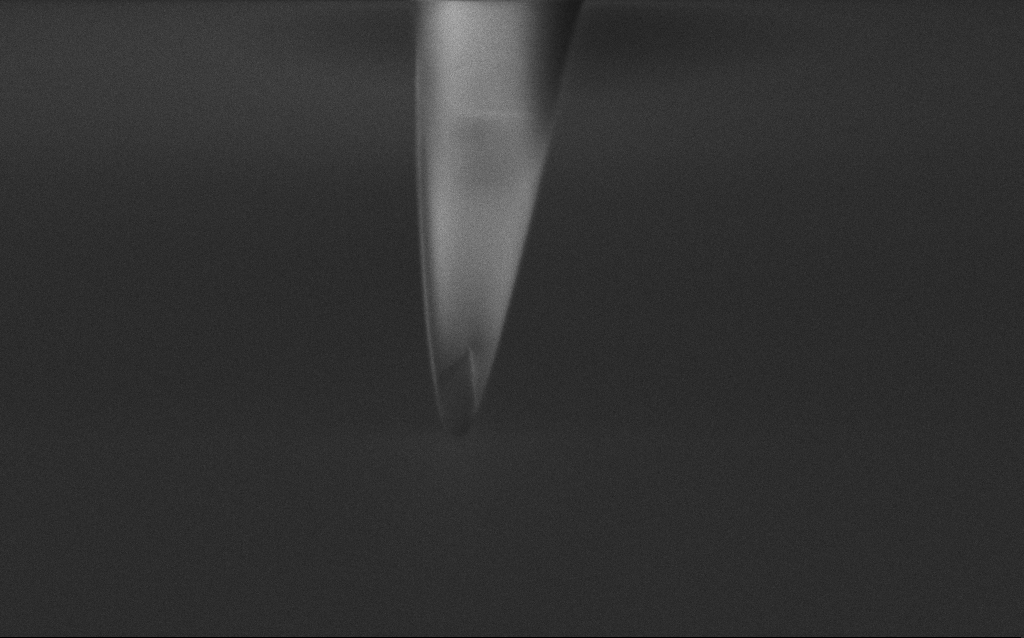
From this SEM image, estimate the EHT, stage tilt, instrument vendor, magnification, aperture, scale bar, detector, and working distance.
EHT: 2 kV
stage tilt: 45°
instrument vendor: Zeiss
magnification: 57.43 K X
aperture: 30 µm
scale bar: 1000 nm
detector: InLens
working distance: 5 mm